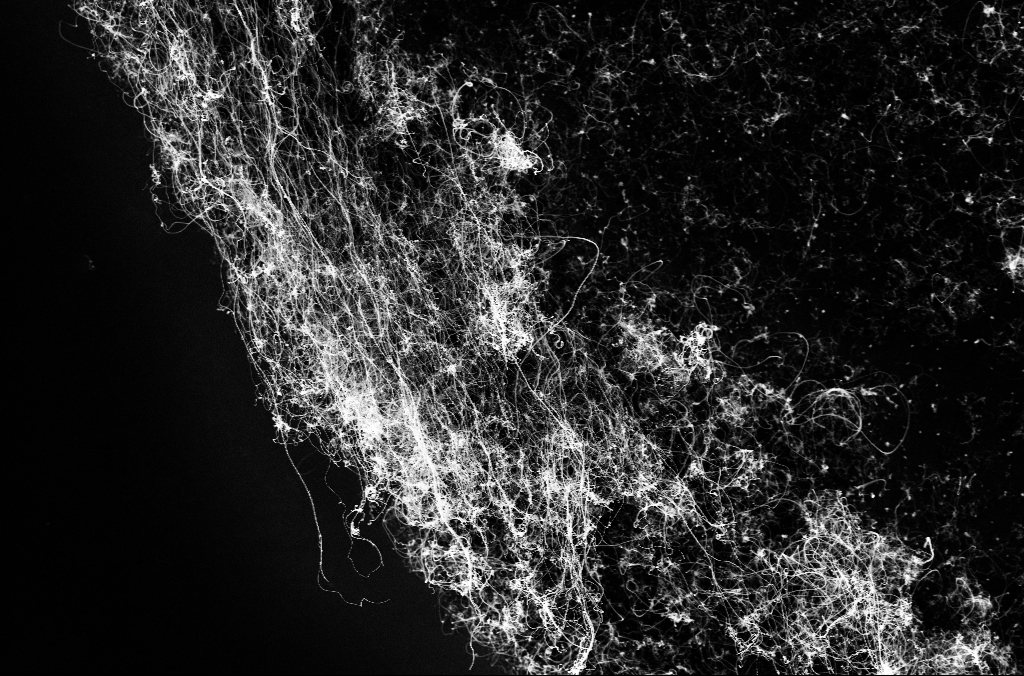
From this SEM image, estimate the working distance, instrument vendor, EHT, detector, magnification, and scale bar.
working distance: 3.3 mm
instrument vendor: Zeiss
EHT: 10 kV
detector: InLens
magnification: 5 K X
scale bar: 10000 nm